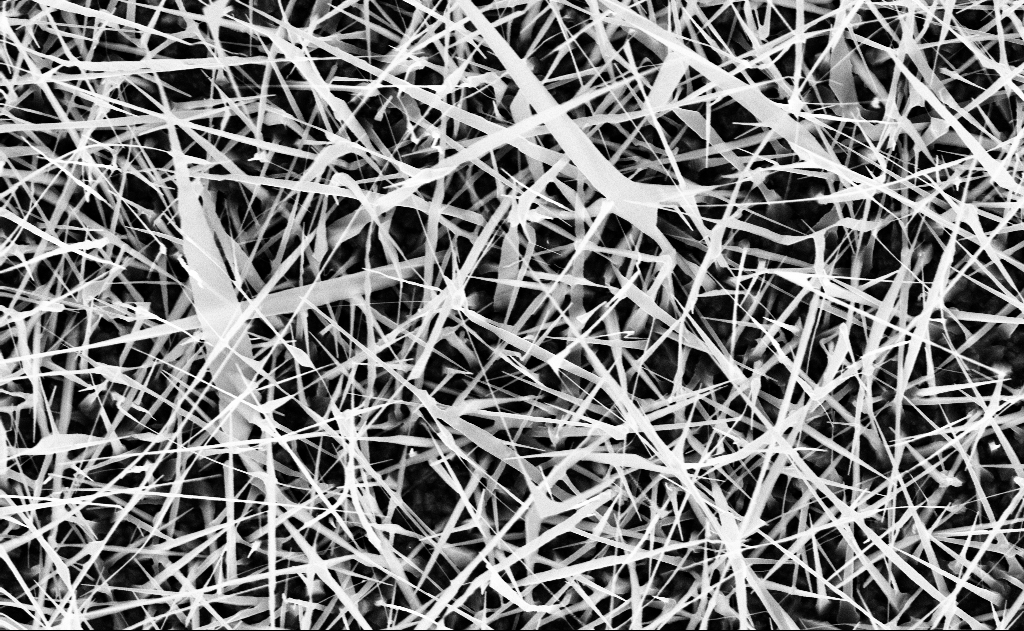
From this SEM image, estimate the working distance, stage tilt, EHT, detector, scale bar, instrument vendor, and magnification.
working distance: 15 mm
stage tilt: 0°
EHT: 10 kV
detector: InLens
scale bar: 2000 nm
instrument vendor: Zeiss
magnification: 20 K X